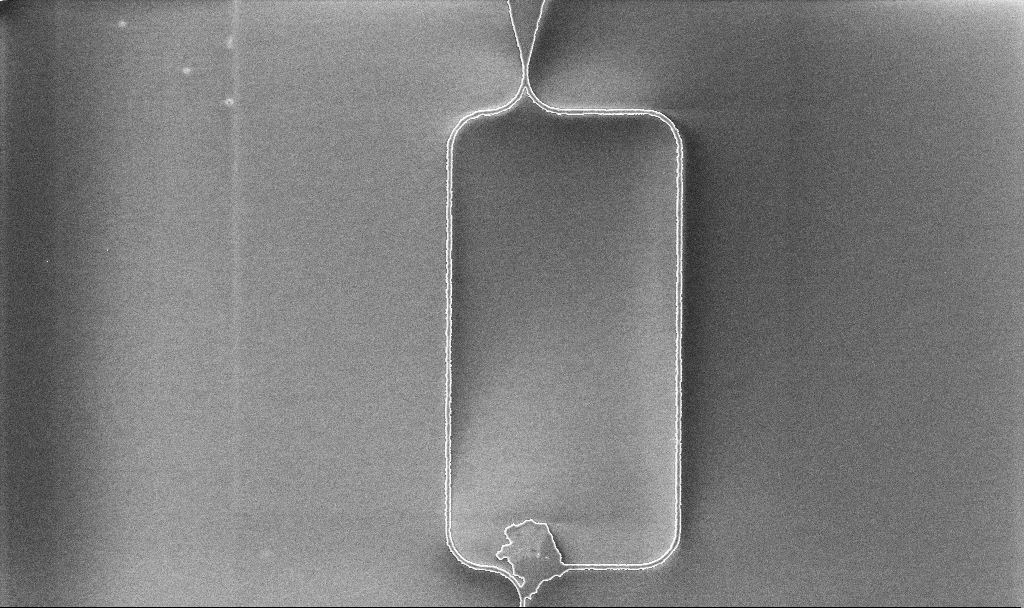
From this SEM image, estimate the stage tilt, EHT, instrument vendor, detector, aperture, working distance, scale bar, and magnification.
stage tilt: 0°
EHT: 5 kV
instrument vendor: Zeiss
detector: InLens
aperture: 30 µm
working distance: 10.1 mm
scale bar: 10000 nm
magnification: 2.94 K X